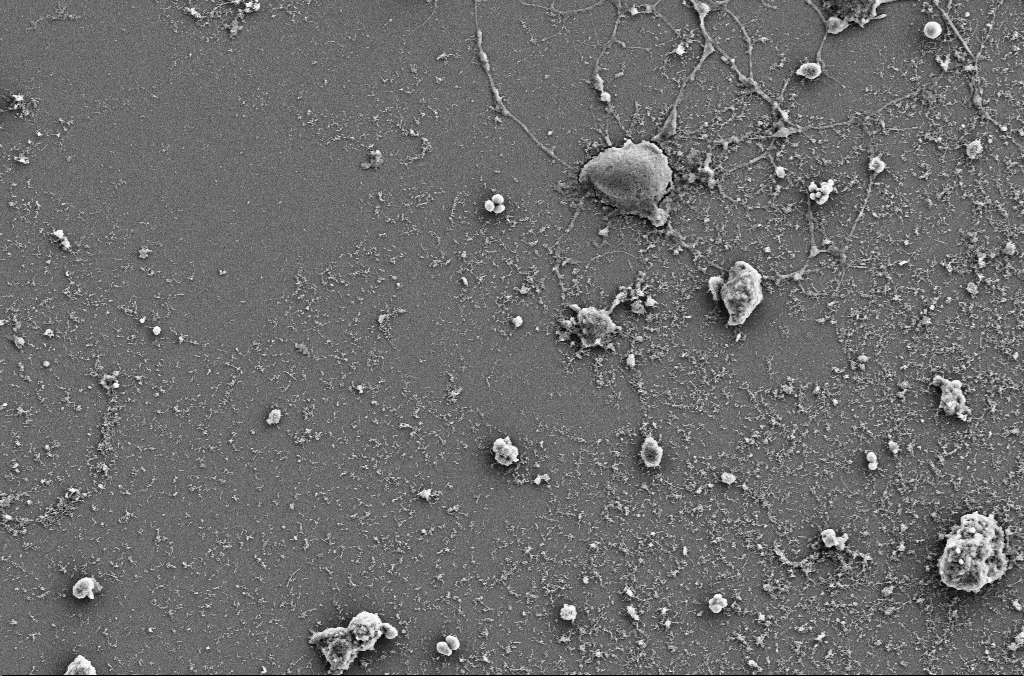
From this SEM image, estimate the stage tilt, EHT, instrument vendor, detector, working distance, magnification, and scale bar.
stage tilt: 0°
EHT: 5 kV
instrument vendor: Zeiss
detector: SE2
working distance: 4 mm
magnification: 4 K X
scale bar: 10000 nm